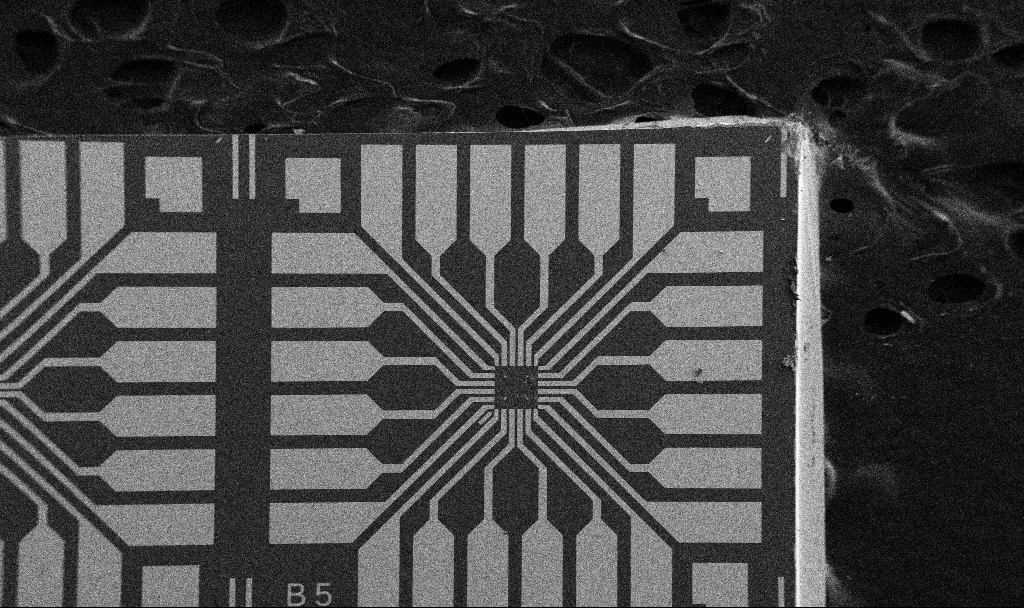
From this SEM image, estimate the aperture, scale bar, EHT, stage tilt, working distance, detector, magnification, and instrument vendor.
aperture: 30 µm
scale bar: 200000 nm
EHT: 5 kV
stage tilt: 0°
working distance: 10.7 mm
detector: SE2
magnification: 0.1 K X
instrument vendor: Zeiss